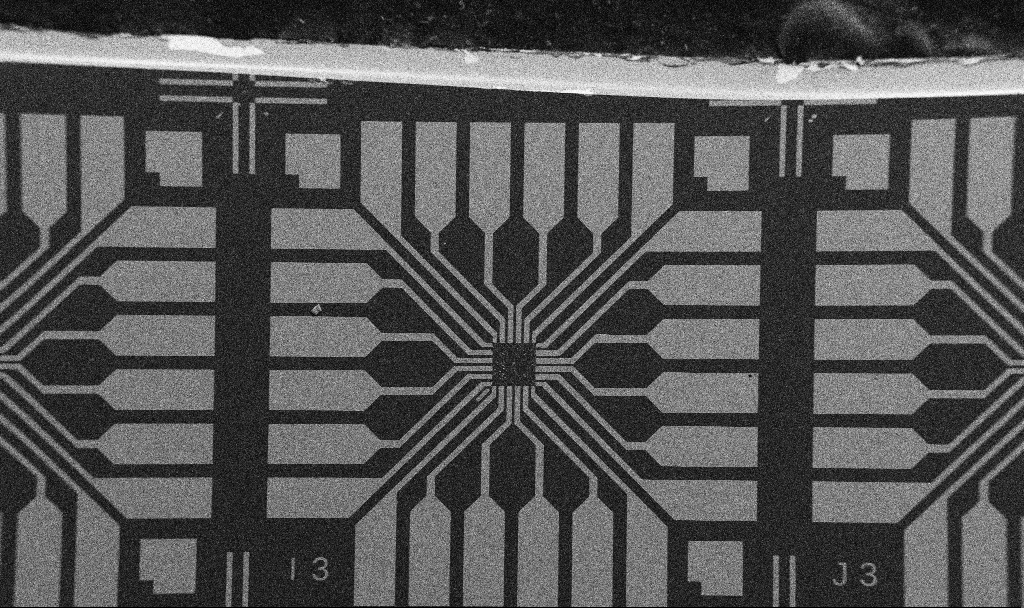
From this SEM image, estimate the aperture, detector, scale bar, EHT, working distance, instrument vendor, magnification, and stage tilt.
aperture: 30 µm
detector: SE2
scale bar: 200000 nm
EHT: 5 kV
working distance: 10.7 mm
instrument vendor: Zeiss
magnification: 0.1 K X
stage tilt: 0°